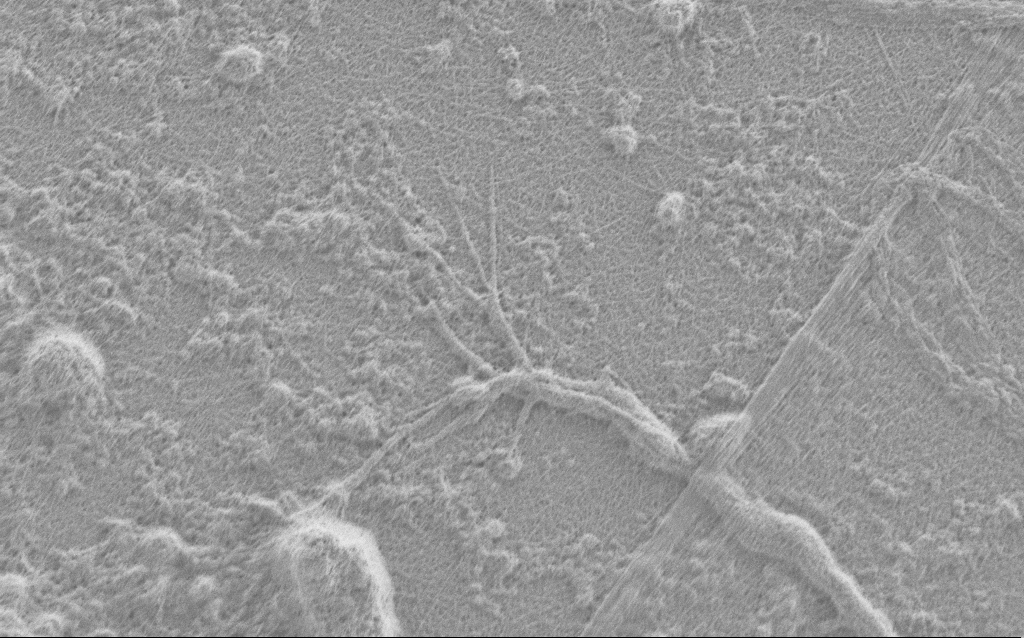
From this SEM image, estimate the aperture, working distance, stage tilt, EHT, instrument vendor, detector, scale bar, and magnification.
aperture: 30 µm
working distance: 4 mm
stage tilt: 0°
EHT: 0.9 kV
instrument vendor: Zeiss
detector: SE2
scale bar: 2000 nm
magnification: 10 K X